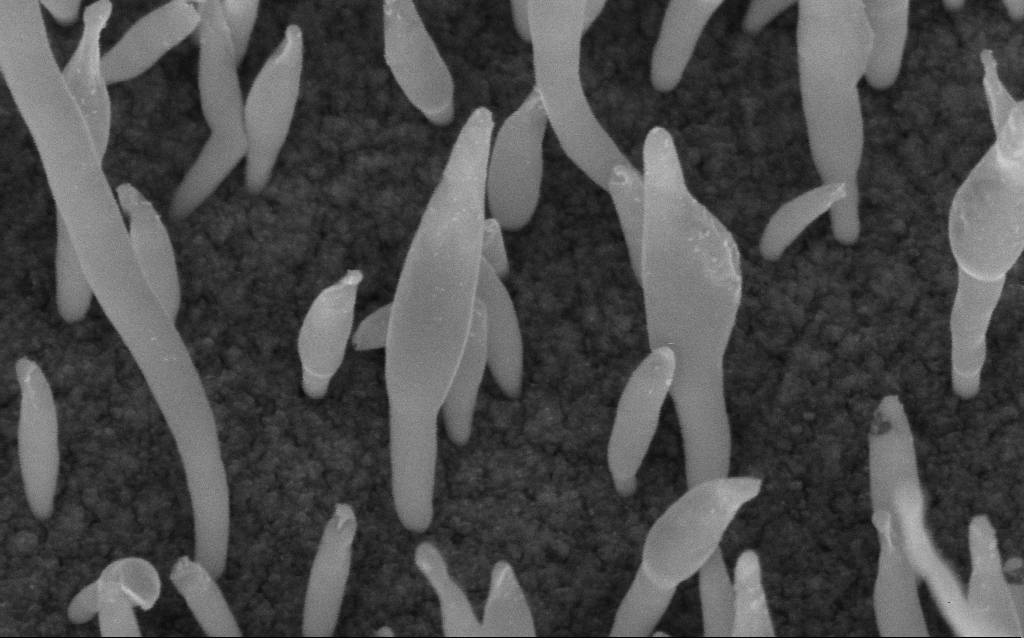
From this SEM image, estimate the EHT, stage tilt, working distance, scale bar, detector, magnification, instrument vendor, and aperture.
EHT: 5 kV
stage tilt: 45°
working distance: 6.4 mm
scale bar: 100 nm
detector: InLens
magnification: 200 K X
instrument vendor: Zeiss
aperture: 30 µm